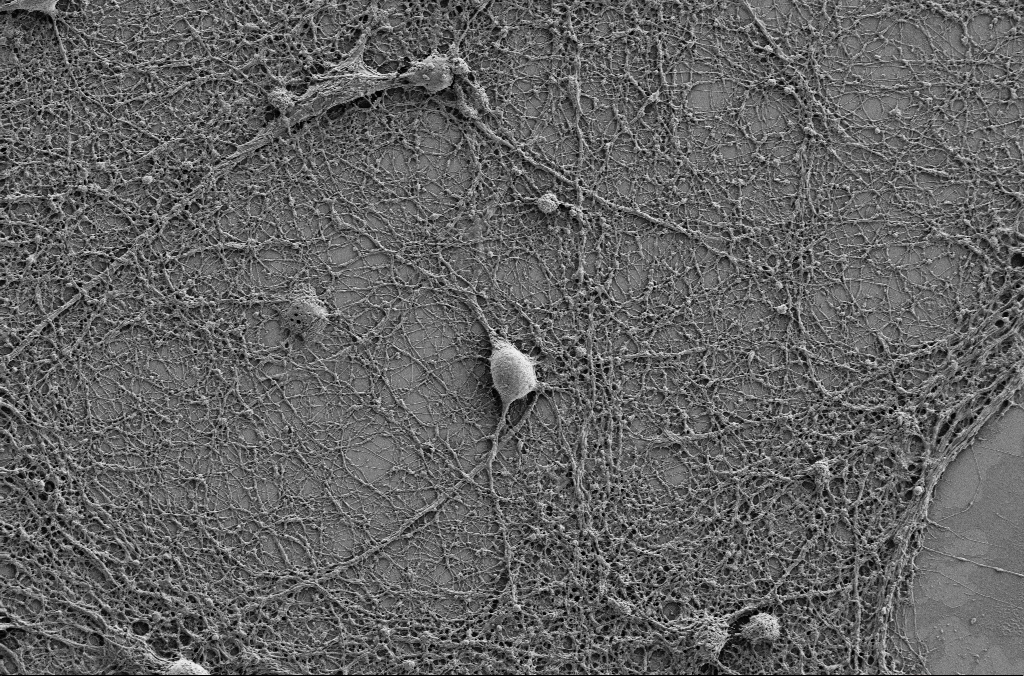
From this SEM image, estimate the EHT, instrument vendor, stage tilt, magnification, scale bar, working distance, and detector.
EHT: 1 kV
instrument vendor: Zeiss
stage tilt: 0°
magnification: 2.5 K X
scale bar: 10000 nm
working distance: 4.1 mm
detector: SE2